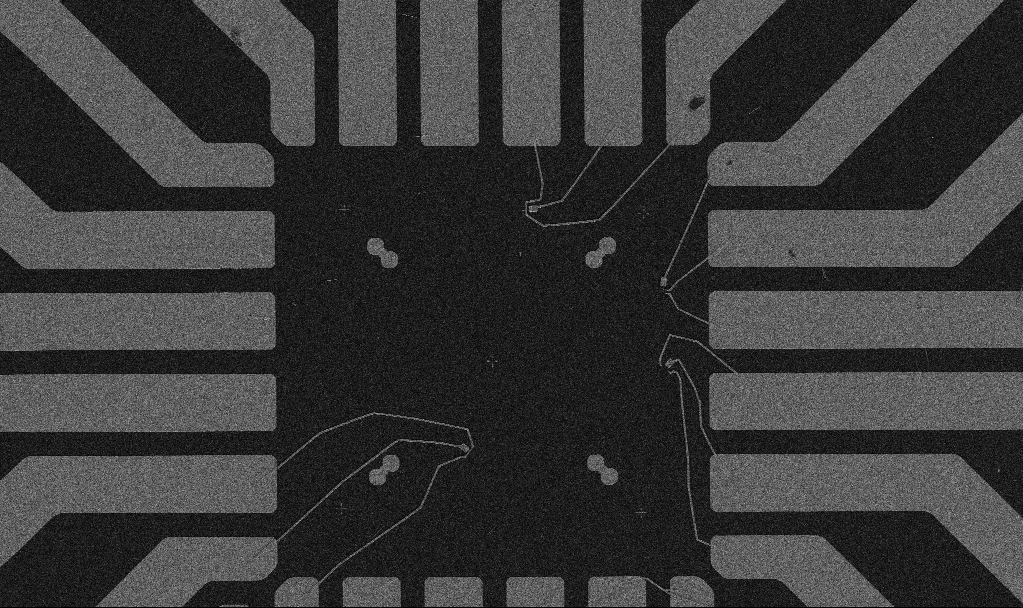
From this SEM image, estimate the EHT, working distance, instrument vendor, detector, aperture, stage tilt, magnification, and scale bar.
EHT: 5 kV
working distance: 10.4 mm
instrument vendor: Zeiss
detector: SE2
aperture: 30 µm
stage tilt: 0°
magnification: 1 K X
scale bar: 20000 nm